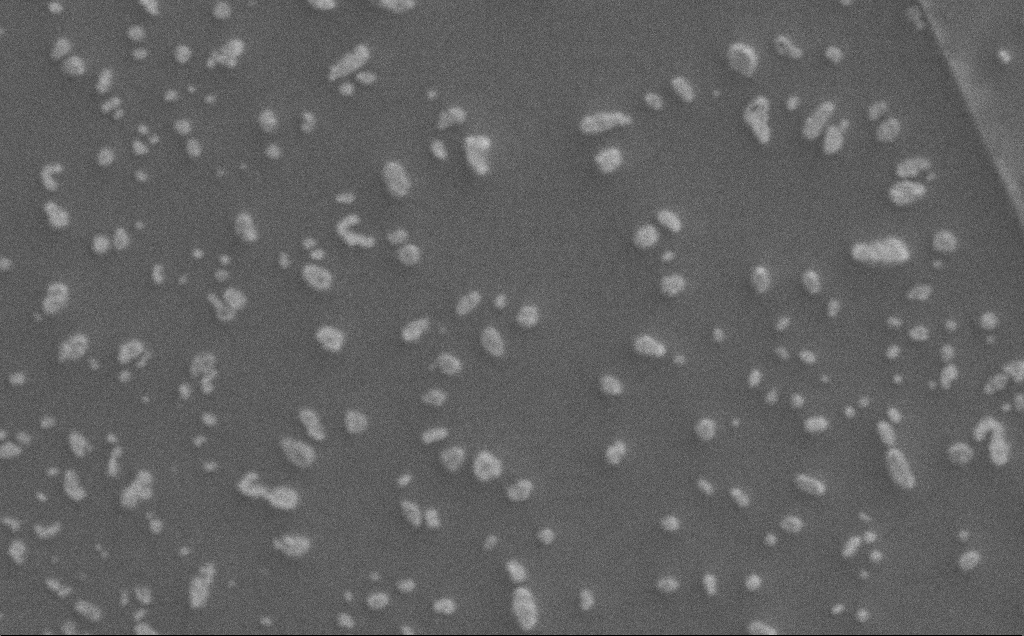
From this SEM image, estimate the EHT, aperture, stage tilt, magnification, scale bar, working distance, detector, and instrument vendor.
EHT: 1 kV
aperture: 30 µm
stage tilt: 0°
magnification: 6.9 K X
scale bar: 10000 nm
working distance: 3 mm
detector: SE2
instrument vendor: Zeiss